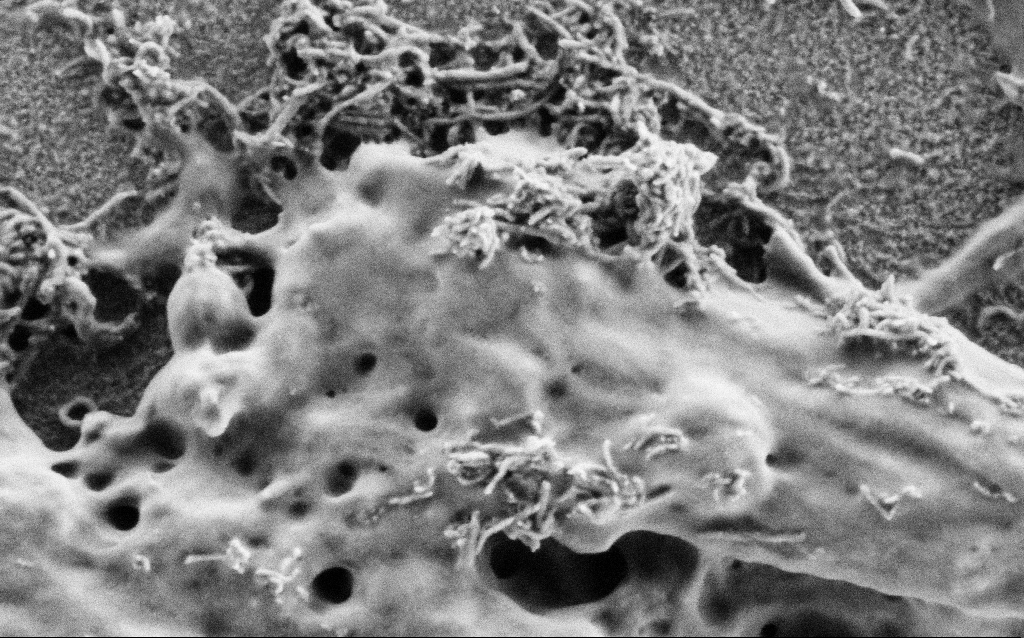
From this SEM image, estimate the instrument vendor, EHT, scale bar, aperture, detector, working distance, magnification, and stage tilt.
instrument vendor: Zeiss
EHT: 1 kV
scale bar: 200 nm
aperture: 30 µm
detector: SE2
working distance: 4 mm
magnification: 100 K X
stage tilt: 0°